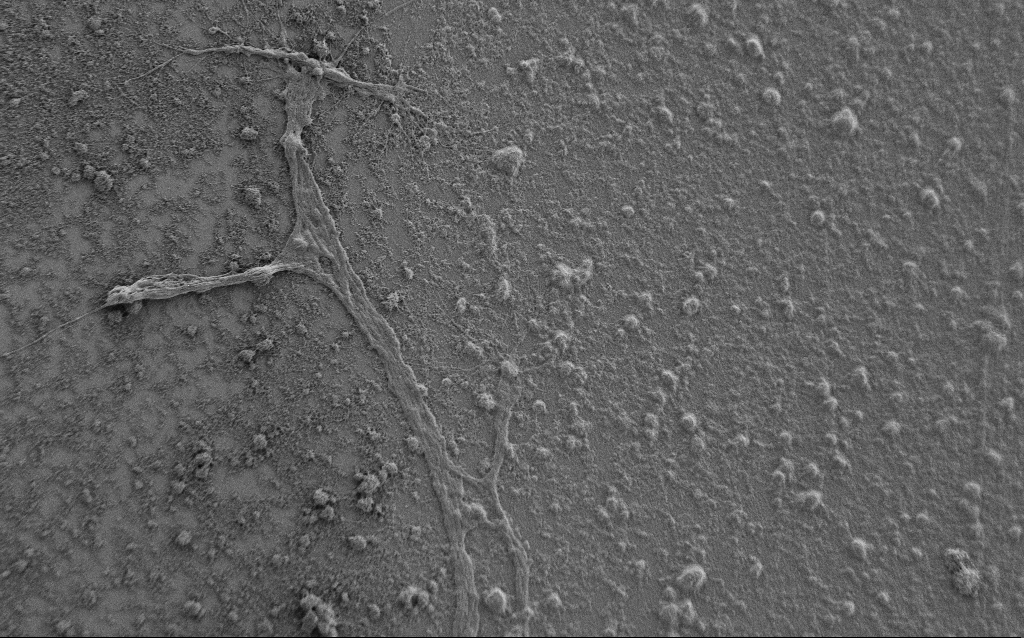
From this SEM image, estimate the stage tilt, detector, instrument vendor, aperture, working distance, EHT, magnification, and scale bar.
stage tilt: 0°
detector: SE2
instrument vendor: Zeiss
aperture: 30 µm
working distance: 7 mm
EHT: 0.9 kV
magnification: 2.5 K X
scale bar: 10000 nm